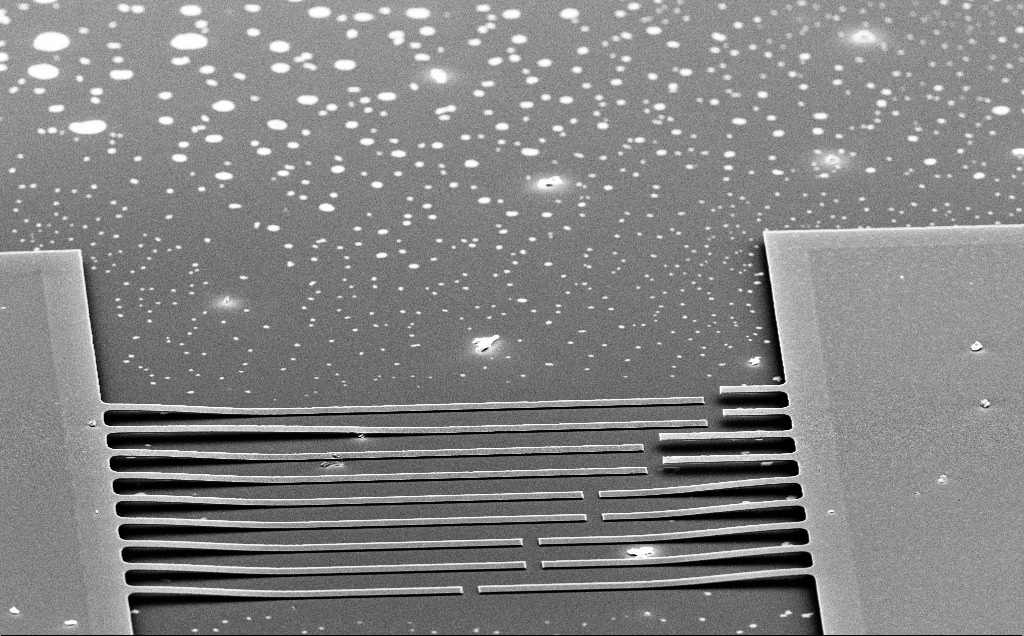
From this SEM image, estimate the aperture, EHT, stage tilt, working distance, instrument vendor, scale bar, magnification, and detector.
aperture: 30 µm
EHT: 10 kV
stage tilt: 65.4°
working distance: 18 mm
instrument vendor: Zeiss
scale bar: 20000 nm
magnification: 0.982 K X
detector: SE2